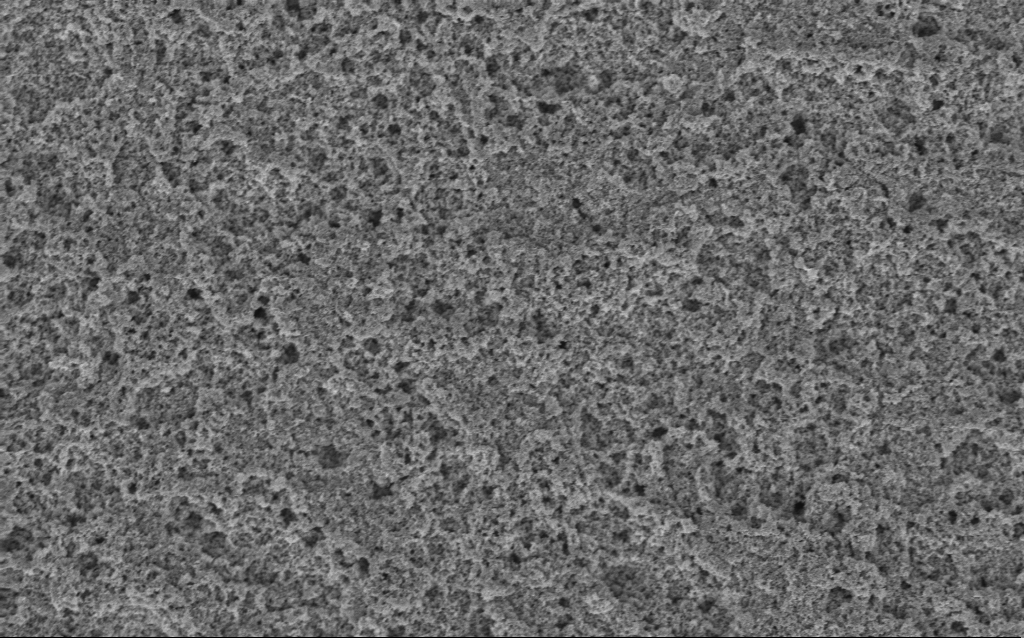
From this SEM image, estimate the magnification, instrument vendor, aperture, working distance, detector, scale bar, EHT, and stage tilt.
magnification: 15.33 K X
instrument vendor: Zeiss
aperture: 30 µm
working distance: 10 mm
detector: InLens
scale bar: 1000 nm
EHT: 3 kV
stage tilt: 0°